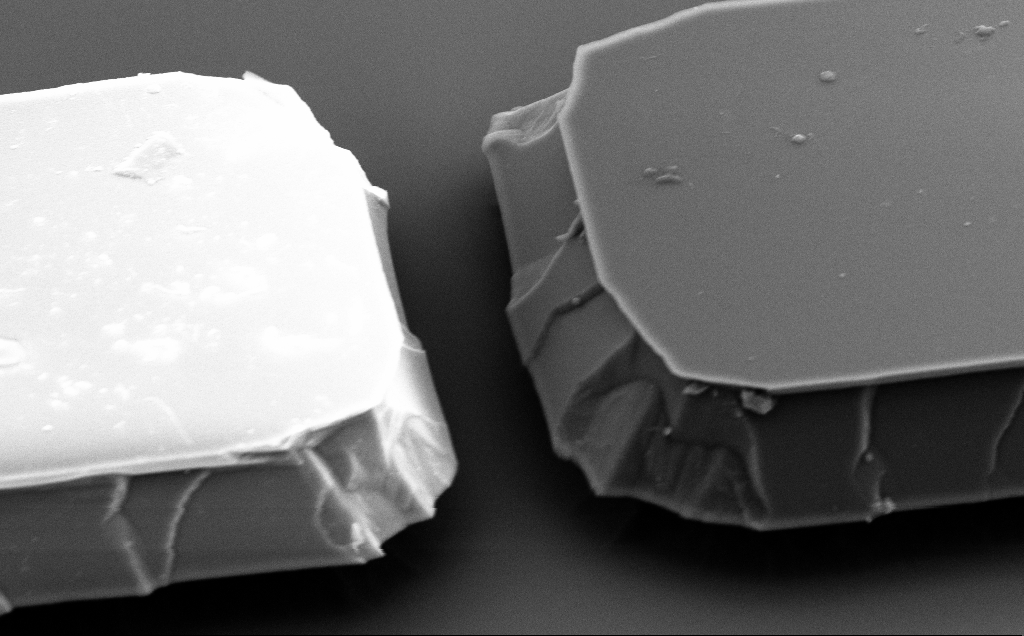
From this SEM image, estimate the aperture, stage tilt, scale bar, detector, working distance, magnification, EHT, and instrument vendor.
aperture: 30 µm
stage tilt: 50°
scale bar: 2000 nm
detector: SE2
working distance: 10 mm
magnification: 14.31 K X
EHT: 5 kV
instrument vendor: Zeiss